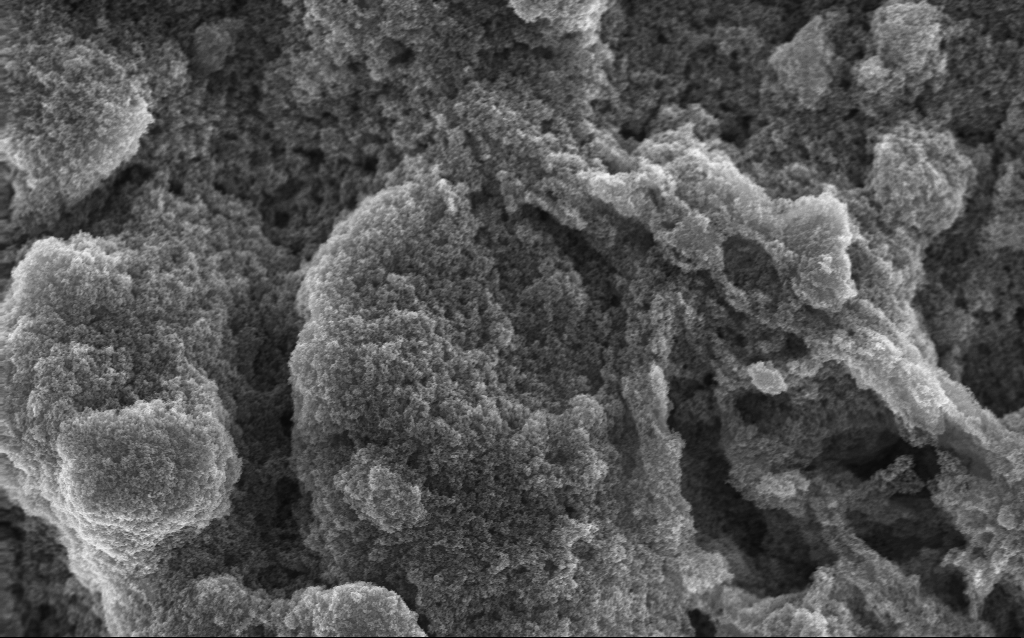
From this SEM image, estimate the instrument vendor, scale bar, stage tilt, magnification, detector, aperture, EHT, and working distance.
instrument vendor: Zeiss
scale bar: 1000 nm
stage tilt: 0°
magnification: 20.87 K X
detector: InLens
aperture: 30 µm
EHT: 10 kV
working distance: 2.8 mm